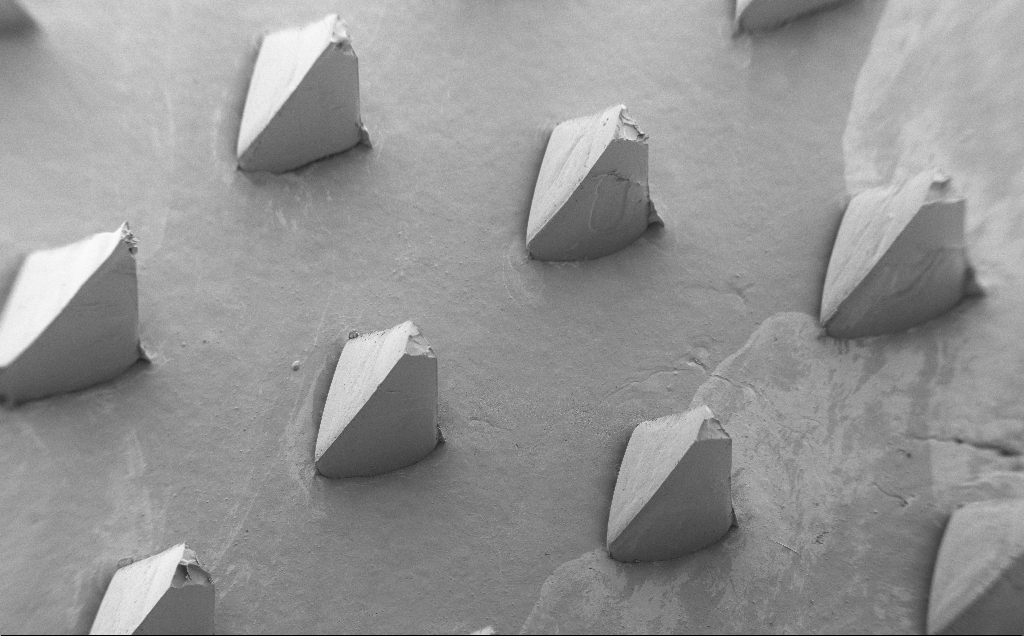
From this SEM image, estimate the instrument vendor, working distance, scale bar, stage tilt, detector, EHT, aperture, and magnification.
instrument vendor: Zeiss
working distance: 8 mm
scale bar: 1e+06 nm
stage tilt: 40°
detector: SE2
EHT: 5 kV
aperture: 30 µm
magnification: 0.071 K X